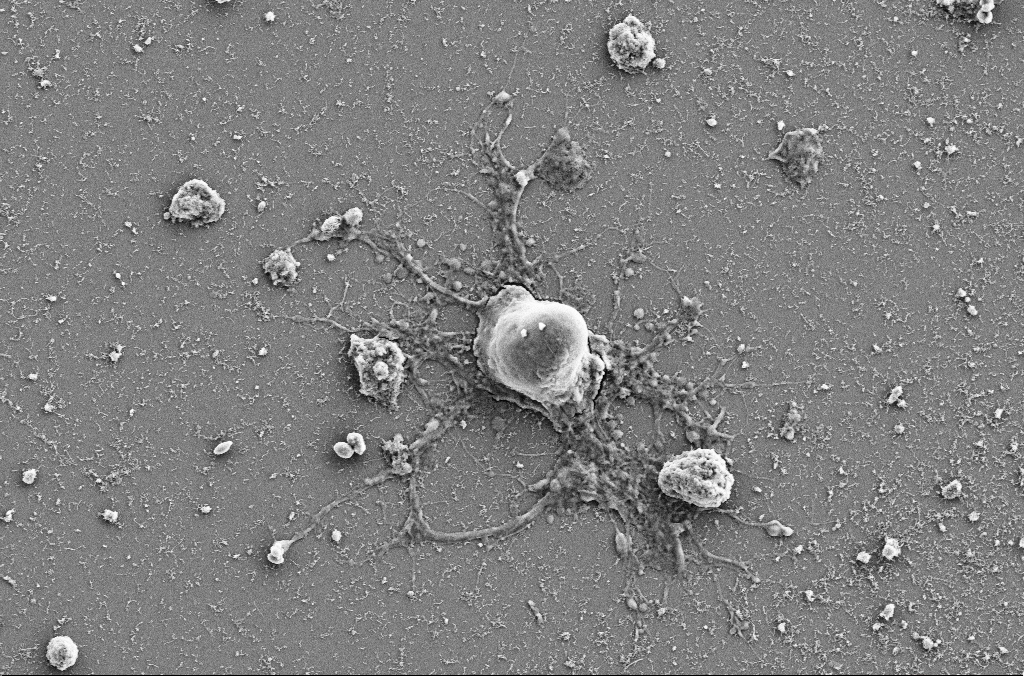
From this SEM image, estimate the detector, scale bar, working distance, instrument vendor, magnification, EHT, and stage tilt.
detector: SE2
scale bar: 10000 nm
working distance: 4 mm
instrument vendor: Zeiss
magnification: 4 K X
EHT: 5 kV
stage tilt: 0°